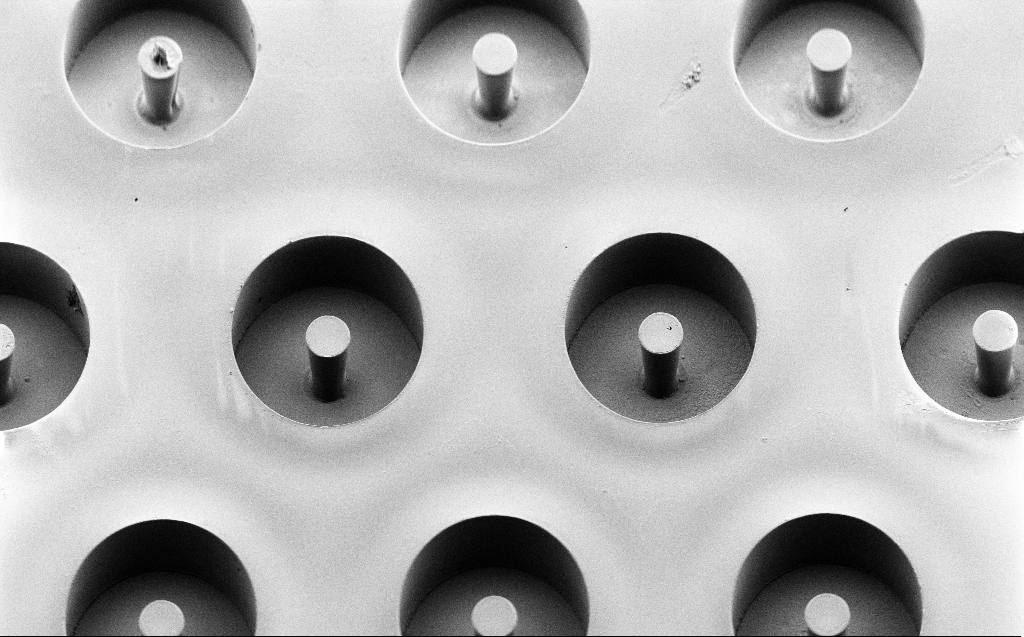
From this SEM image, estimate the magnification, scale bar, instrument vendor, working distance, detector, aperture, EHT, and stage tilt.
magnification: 0.766 K X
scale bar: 20000 nm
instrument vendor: Zeiss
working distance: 7 mm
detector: SE2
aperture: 30 µm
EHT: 2 kV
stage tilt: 45°